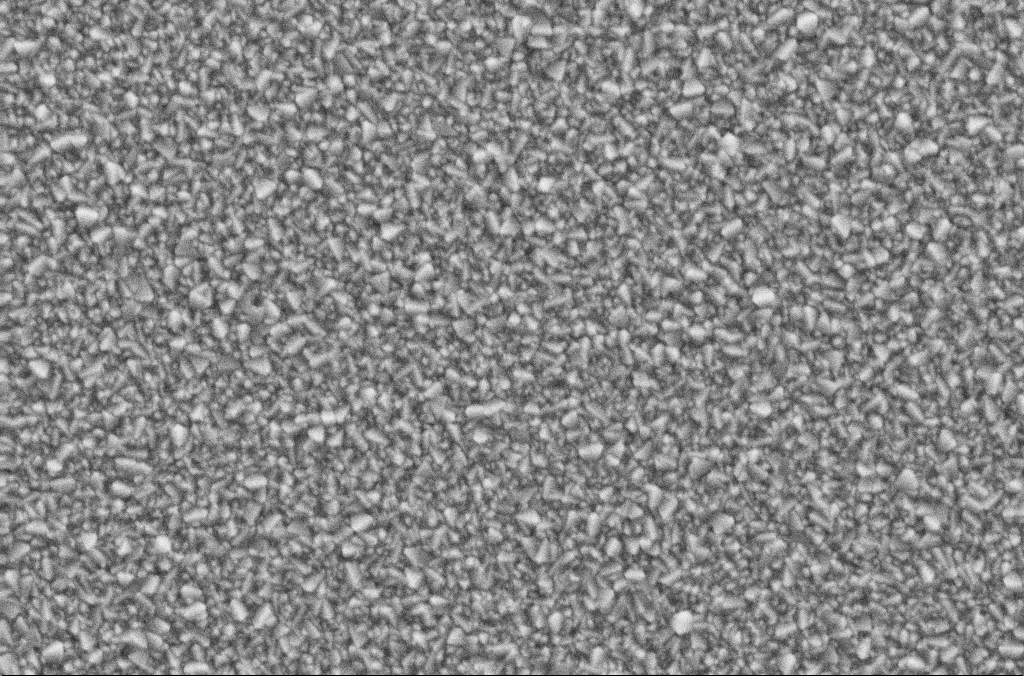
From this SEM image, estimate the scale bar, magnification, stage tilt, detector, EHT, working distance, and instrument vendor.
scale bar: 2000 nm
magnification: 20 K X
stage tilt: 0°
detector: SE2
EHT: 10 kV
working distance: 1.9 mm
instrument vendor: Zeiss